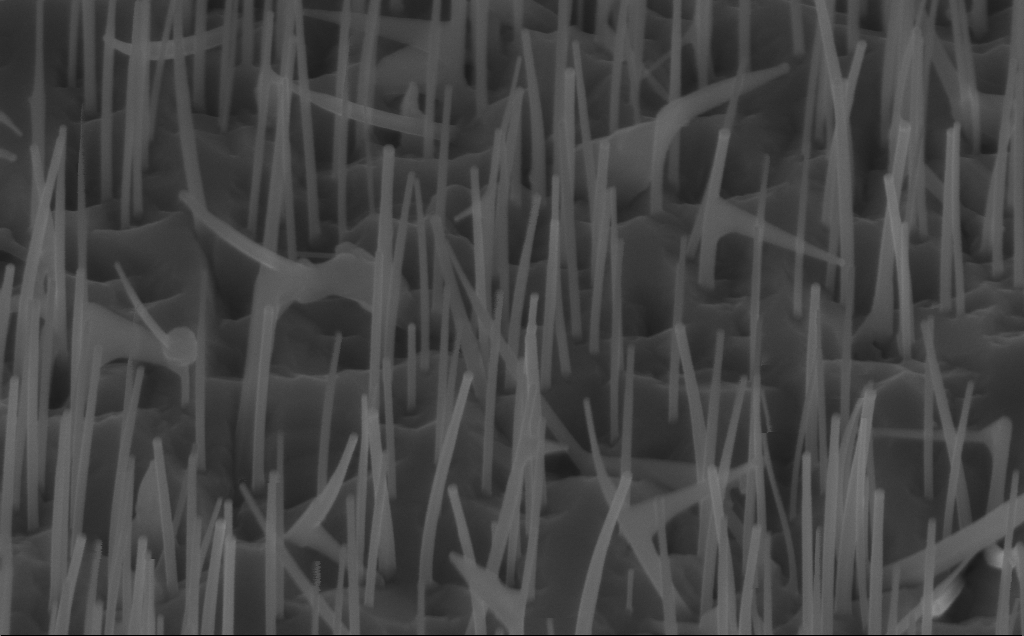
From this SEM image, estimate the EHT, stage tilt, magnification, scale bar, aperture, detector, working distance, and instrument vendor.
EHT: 10 kV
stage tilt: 45°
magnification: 80 K X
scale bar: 200 nm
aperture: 30 µm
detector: InLens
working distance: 7 mm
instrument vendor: Zeiss